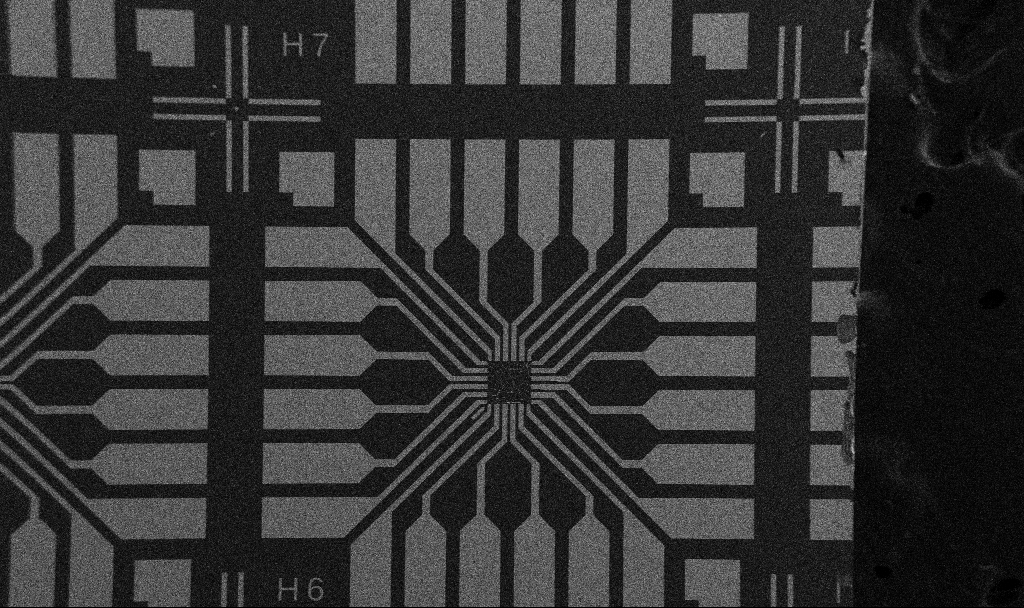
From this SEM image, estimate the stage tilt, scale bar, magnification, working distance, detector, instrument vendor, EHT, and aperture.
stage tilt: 0°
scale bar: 200000 nm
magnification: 0.1 K X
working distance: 10.7 mm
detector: SE2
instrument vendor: Zeiss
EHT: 5 kV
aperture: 30 µm